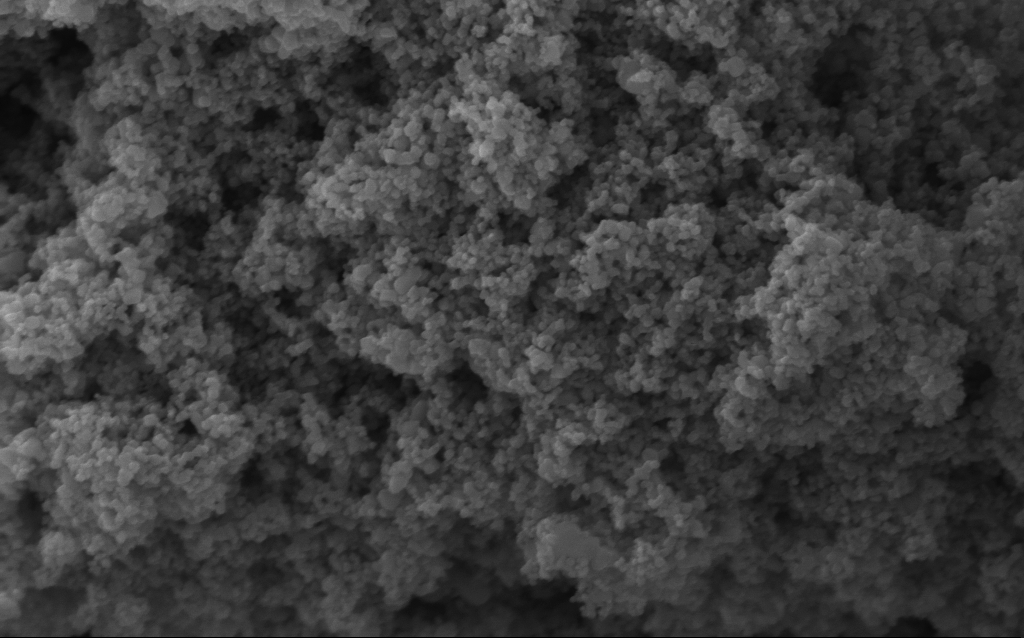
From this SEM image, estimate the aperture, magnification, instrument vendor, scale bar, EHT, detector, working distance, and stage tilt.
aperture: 30 µm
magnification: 114.56 K X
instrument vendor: Zeiss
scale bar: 200 nm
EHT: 5 kV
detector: InLens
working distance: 4.2 mm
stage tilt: -0°